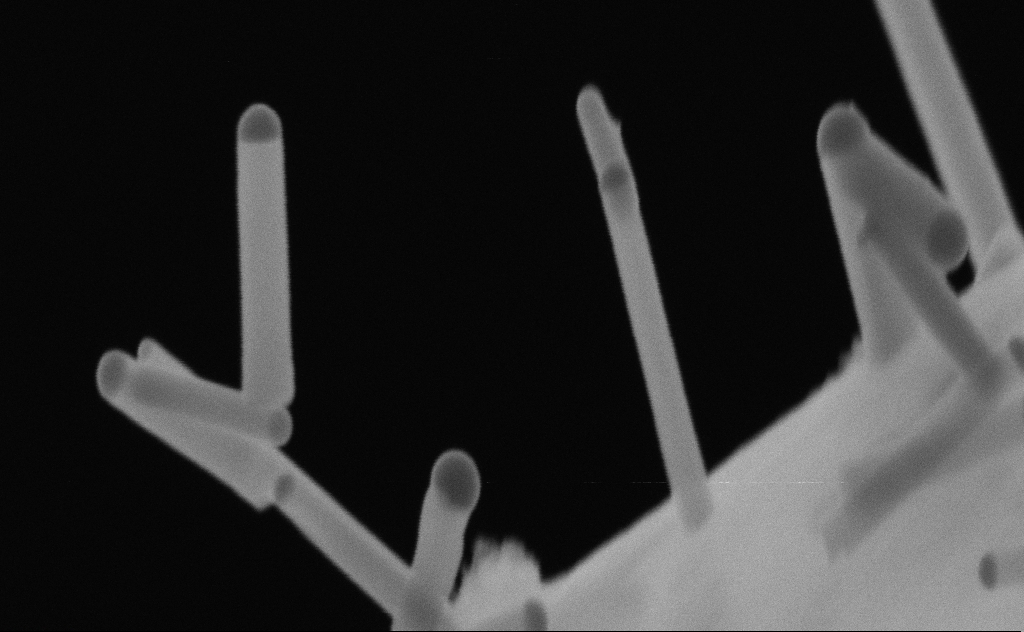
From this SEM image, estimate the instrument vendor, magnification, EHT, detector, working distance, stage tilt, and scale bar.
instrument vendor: Zeiss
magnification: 223.73 K X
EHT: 20 kV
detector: SE2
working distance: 9 mm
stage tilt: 0°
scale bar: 200 nm